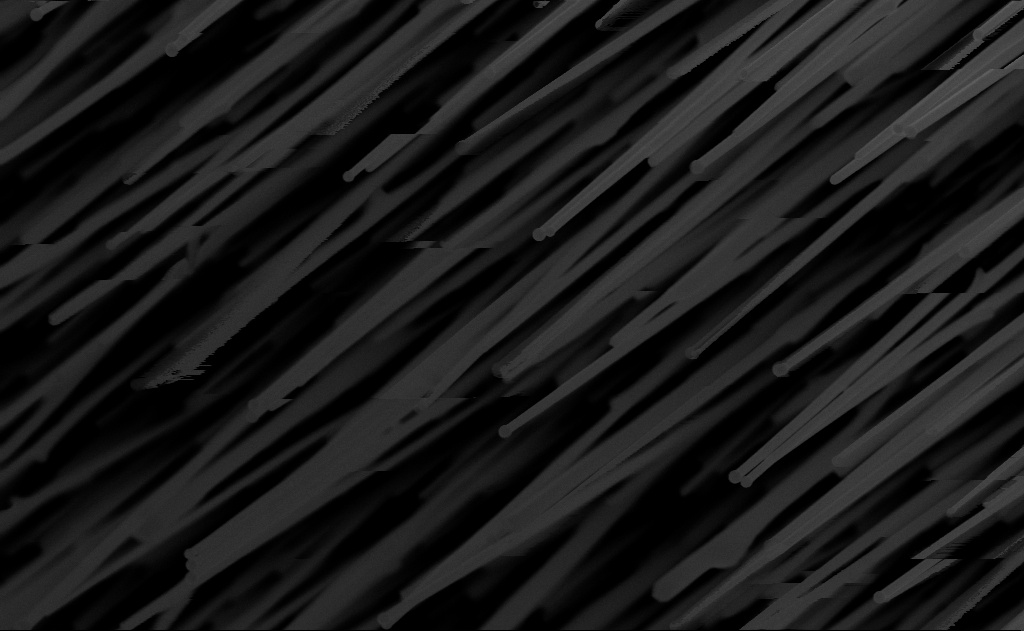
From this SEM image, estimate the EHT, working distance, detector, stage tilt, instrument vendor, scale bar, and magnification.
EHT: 10 kV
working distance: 10 mm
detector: InLens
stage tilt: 0°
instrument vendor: Zeiss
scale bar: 1000 nm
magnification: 48.7 K X